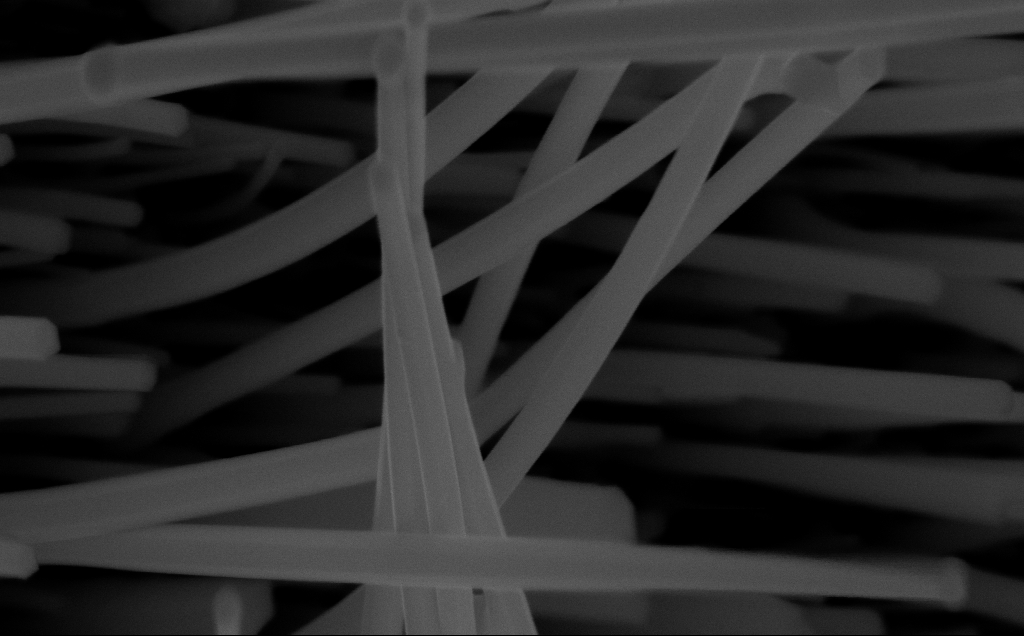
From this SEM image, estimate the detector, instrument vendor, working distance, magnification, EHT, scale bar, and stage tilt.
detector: InLens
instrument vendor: Zeiss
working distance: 6 mm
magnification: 127.42 K X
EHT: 10 kV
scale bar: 200 nm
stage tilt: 0°